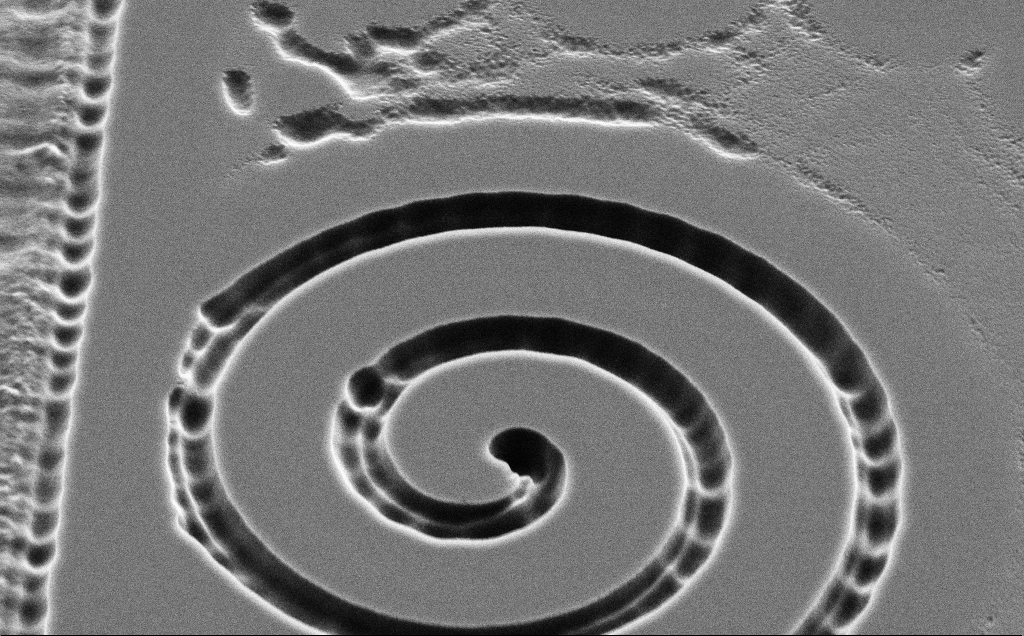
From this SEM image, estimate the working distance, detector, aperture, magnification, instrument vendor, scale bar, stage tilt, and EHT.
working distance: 11 mm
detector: SE2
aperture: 30 µm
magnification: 10.06 K X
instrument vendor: Zeiss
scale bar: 2000 nm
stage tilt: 45°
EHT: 5 kV